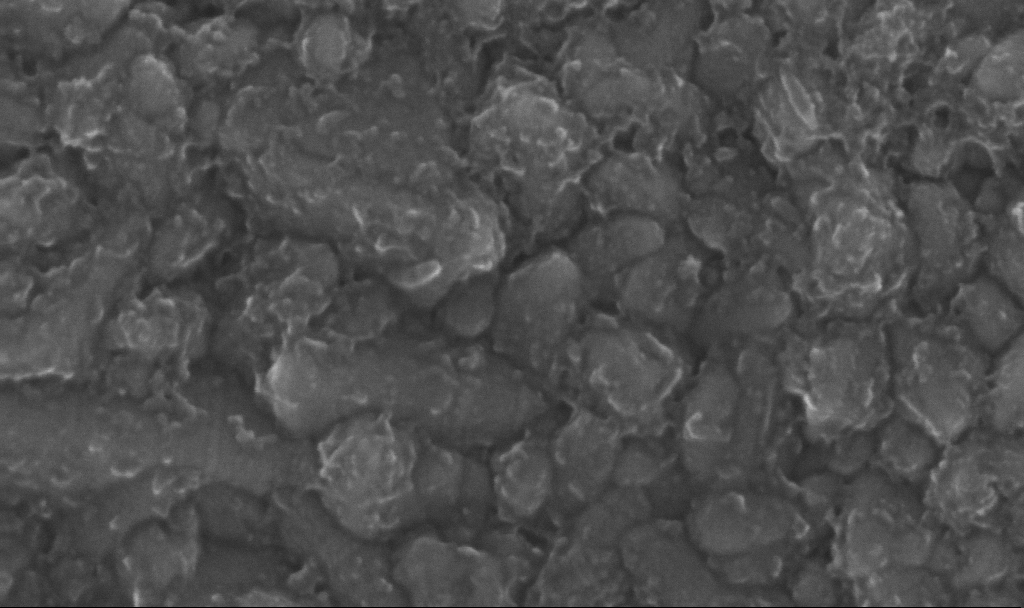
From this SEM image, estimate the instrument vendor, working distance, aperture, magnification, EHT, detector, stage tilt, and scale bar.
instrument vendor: Zeiss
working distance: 4.8 mm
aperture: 30 µm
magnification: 400.61 K X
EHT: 20 kV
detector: InLens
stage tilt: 0°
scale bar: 100 nm